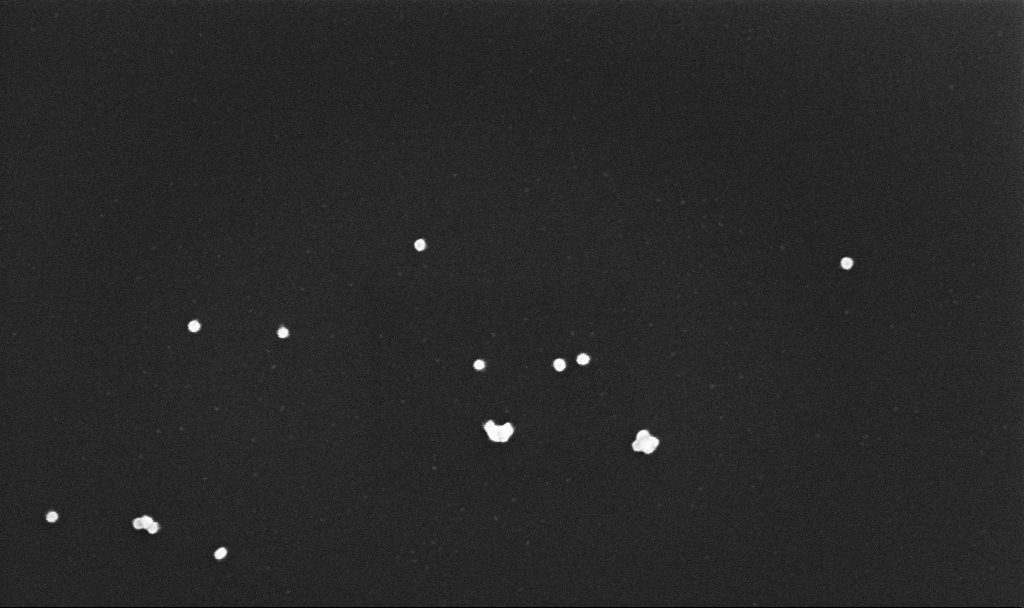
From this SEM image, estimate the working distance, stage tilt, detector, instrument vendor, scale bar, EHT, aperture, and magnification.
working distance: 3.4 mm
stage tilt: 0°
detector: InLens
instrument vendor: Zeiss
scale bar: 100 nm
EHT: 10 kV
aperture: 30 µm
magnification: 200 K X